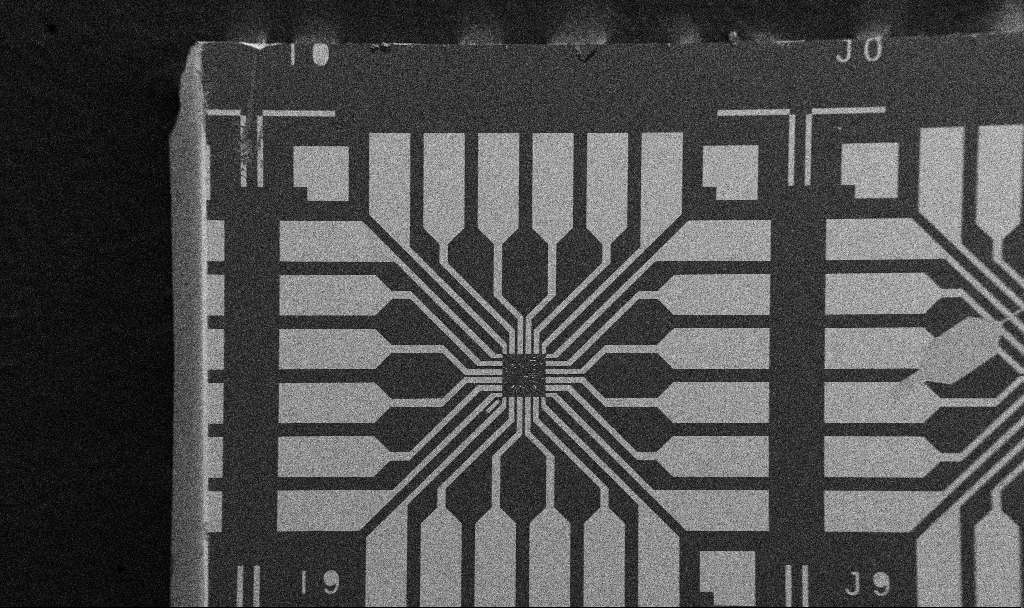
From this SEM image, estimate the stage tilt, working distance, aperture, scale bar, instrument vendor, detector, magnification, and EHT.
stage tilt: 0°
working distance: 10.7 mm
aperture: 30 µm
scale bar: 200000 nm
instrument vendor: Zeiss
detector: SE2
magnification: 0.1 K X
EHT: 5 kV